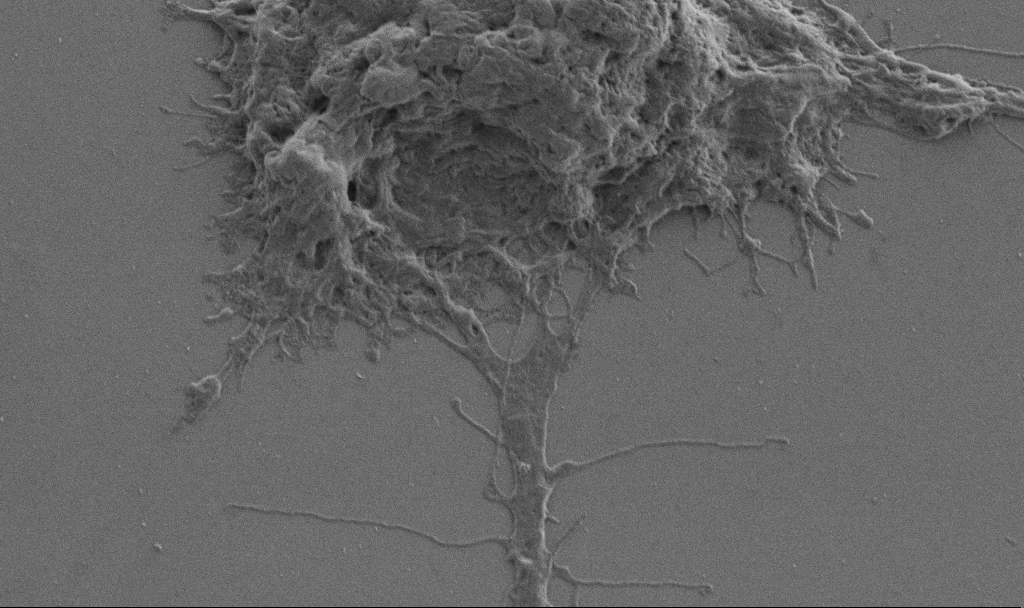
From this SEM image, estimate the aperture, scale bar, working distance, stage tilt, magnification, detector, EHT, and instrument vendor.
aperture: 30 µm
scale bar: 2000 nm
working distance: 6.9 mm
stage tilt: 0°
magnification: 10 K X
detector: SE2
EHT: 1 kV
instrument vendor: Zeiss